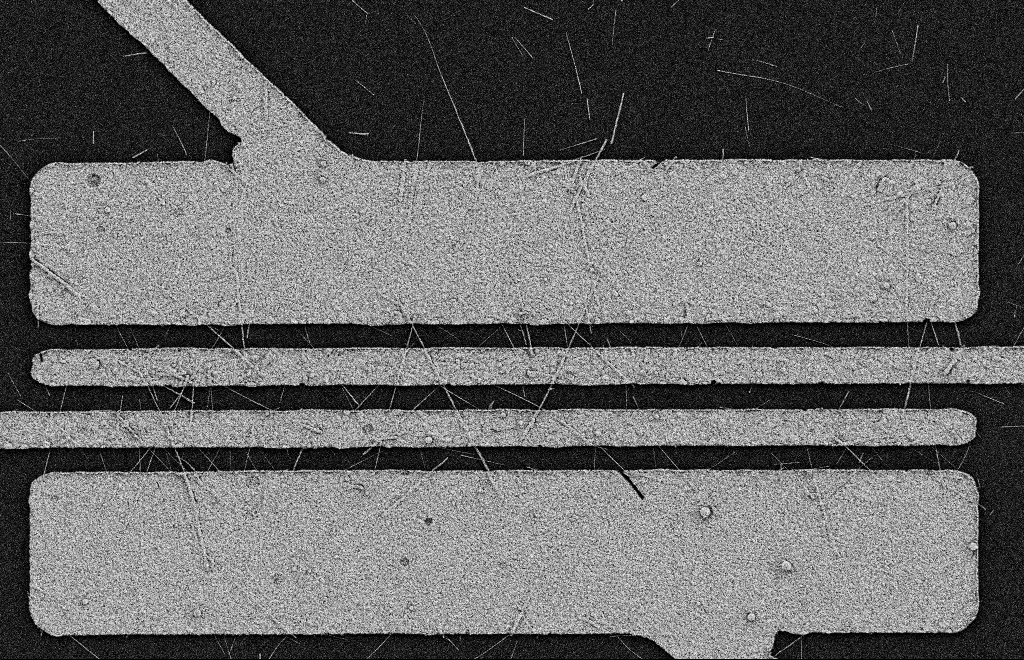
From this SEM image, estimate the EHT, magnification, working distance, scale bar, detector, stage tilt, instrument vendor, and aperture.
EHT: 2 kV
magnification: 5.66 K X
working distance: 11 mm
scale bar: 2000 nm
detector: SE2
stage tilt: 0°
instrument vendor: Zeiss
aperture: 20 µm